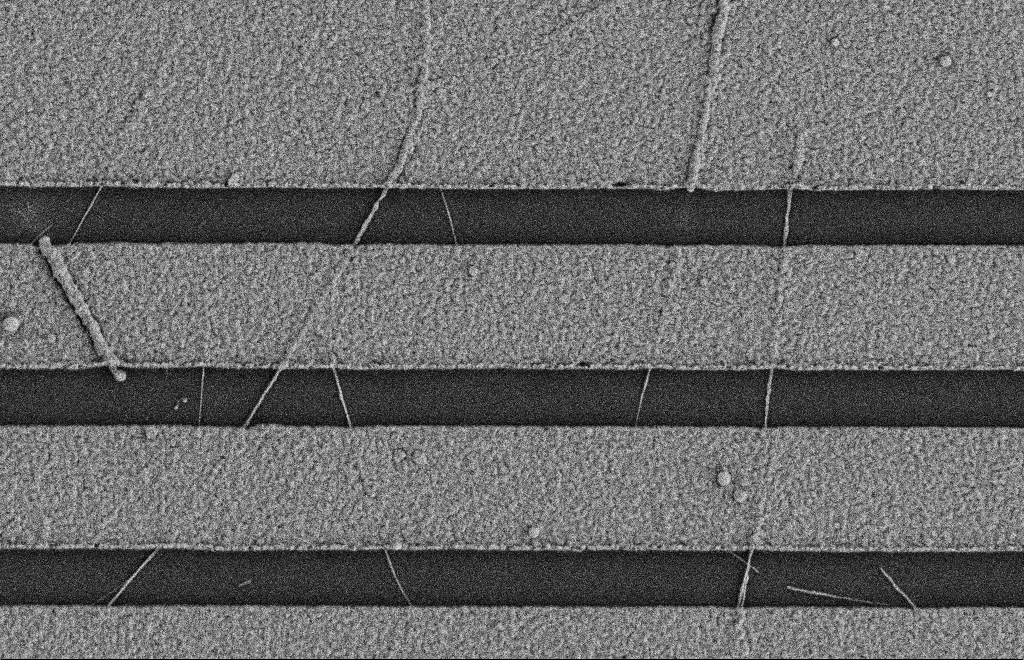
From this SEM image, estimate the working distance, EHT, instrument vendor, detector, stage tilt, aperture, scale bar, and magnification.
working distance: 9 mm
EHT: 2 kV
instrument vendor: Zeiss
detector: SE2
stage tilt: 0°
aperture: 20 µm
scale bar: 2000 nm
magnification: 16.56 K X